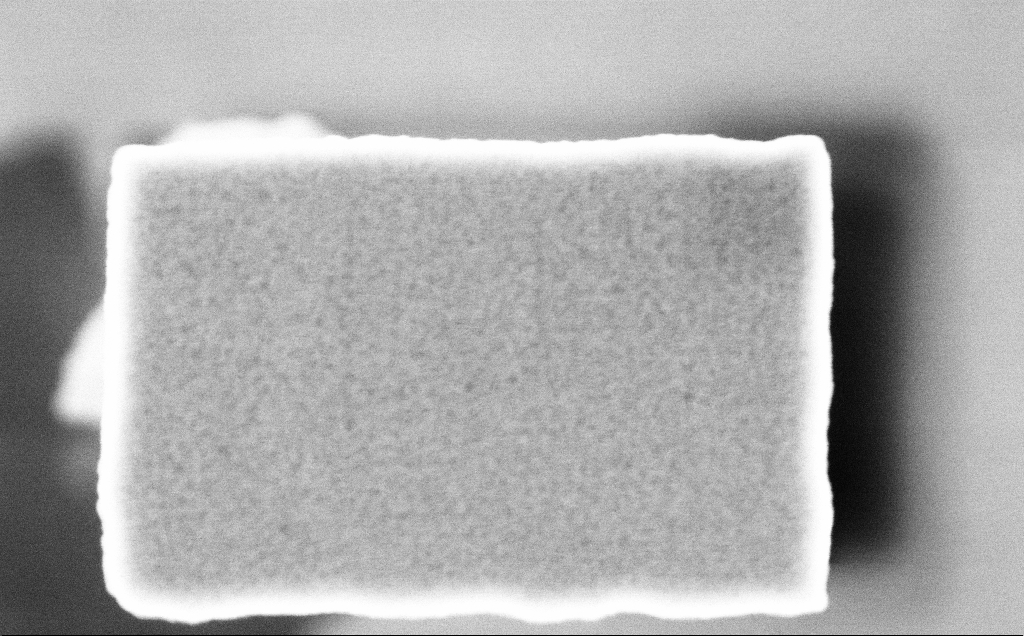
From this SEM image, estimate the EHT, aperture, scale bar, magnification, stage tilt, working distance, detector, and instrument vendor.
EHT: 3 kV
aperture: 30 µm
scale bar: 200 nm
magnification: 90.19 K X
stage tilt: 0°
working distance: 3.2 mm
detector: InLens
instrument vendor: Zeiss